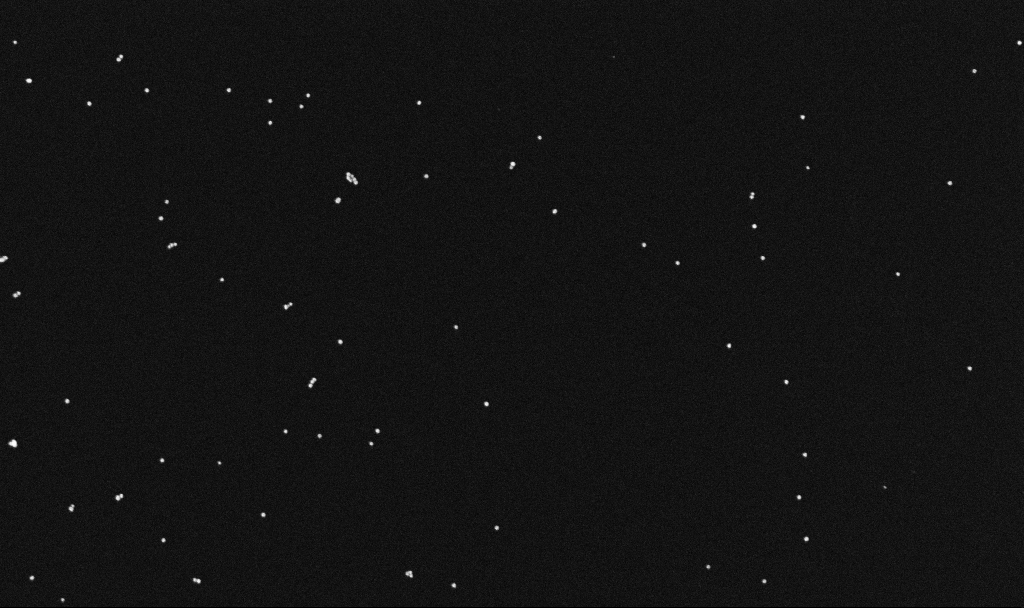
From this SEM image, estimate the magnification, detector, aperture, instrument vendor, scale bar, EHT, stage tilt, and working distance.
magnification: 100 K X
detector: InLens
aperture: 30 µm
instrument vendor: Zeiss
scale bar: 200 nm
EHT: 10 kV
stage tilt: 0°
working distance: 3.4 mm